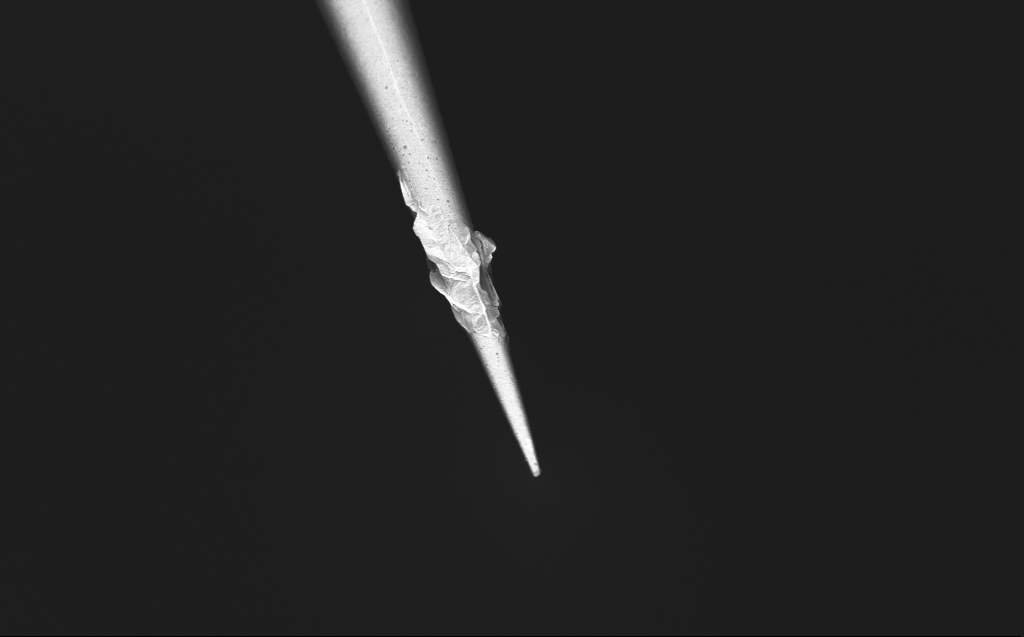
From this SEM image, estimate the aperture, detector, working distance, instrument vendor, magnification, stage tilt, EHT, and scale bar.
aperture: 30 µm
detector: InLens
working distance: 4 mm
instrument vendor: Zeiss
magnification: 5 K X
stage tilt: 45°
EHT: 0.8 kV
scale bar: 10000 nm